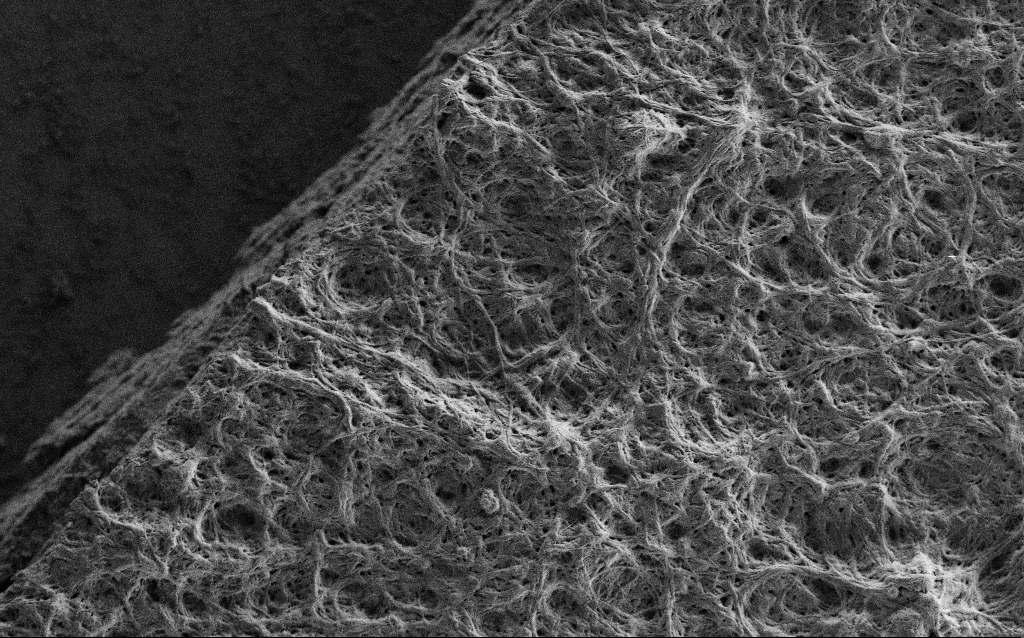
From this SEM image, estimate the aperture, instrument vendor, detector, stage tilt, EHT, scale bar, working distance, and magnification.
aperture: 30 µm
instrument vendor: Zeiss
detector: SE2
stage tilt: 0°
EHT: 0.9 kV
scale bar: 10000 nm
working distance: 4 mm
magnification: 5 K X